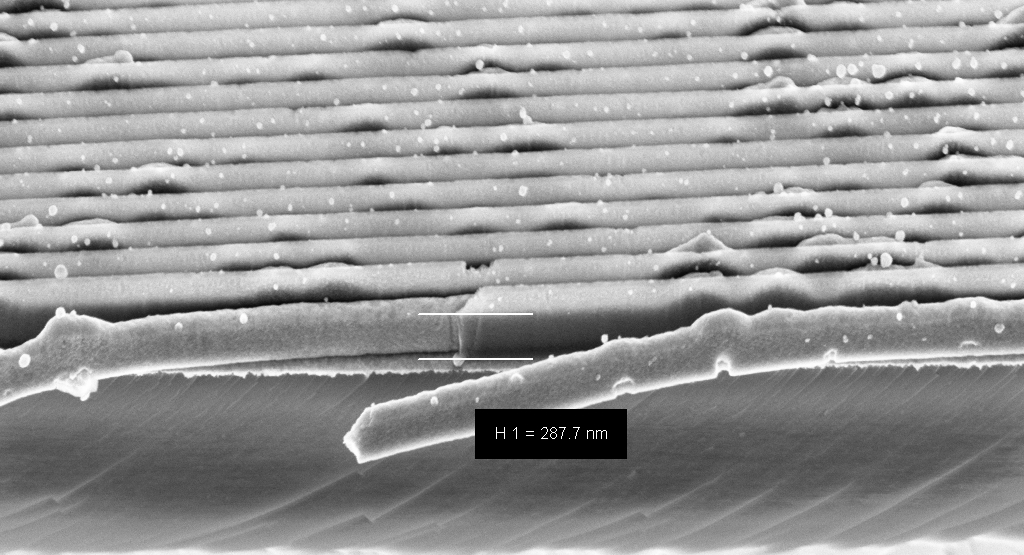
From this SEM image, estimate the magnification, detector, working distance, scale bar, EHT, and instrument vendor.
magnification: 57.44 K X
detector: InLens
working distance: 5 mm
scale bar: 1000 nm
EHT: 5 kV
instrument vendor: Zeiss